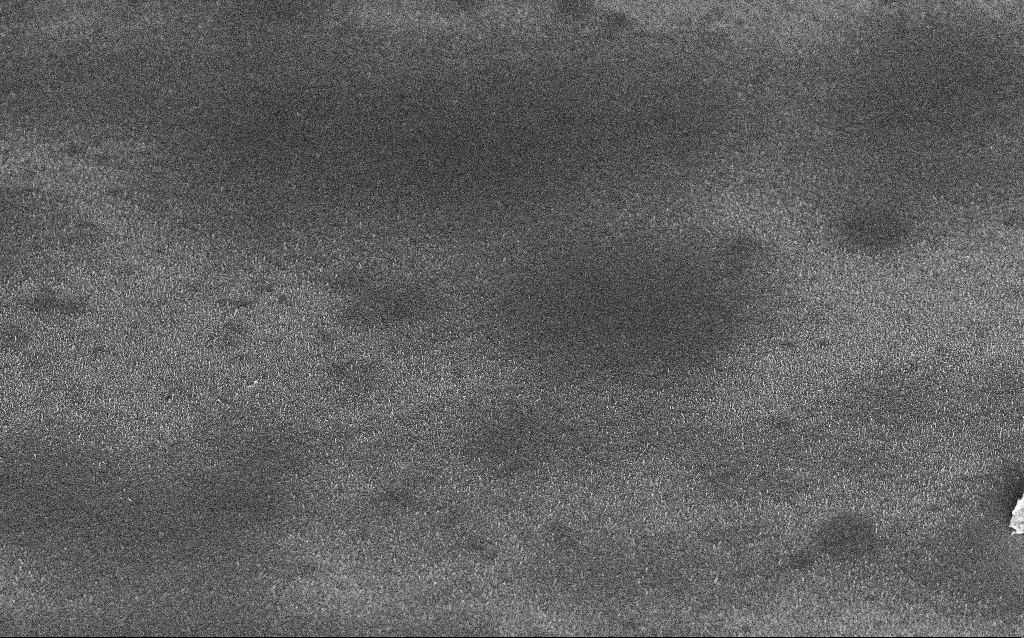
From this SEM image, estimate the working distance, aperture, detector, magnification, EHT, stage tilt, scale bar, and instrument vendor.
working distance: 6.7 mm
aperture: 30 µm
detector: InLens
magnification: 2.46 K X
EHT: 5 kV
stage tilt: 45°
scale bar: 10000 nm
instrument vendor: Zeiss